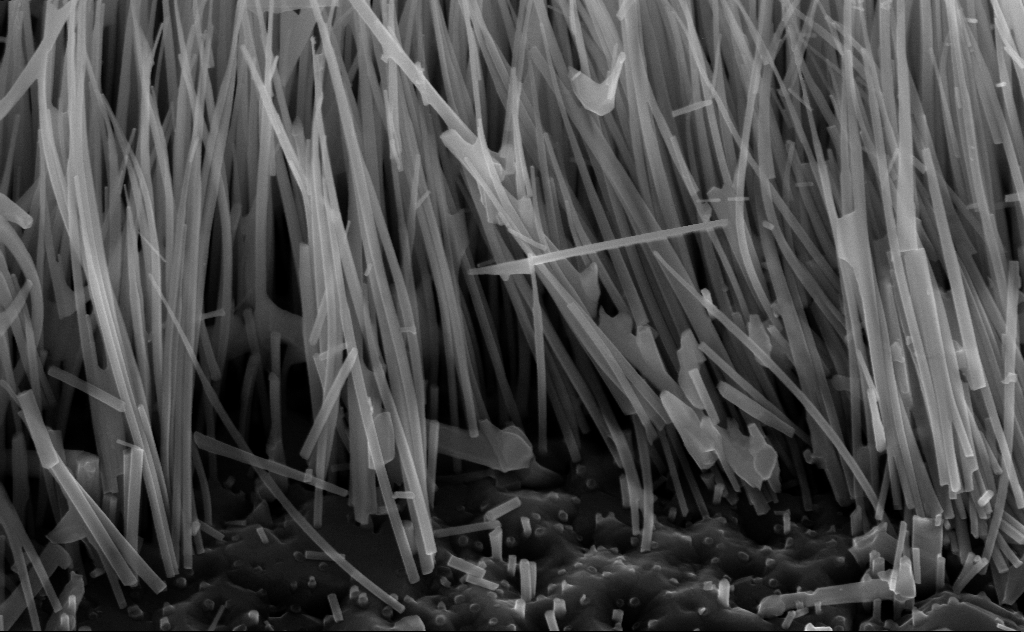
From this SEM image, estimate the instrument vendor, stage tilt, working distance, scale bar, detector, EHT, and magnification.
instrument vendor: Zeiss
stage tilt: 45°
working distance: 6 mm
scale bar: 200 nm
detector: InLens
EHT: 10 kV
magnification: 80 K X